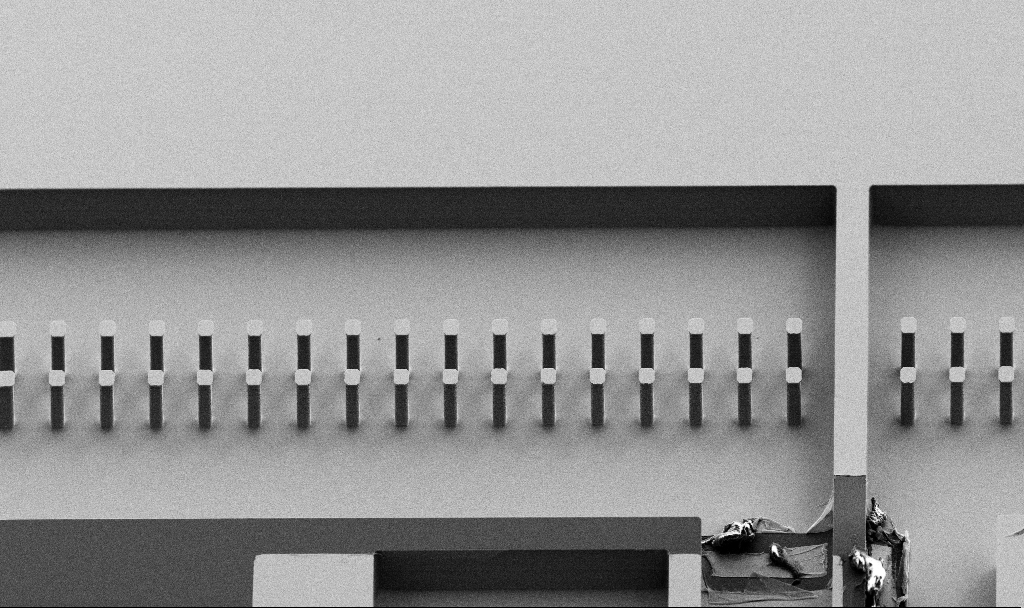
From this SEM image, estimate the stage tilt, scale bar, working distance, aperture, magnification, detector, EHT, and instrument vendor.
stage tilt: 45°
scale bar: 100000 nm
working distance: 8.2 mm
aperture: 30 µm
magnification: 0.6 K X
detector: SE2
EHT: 5 kV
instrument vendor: Zeiss